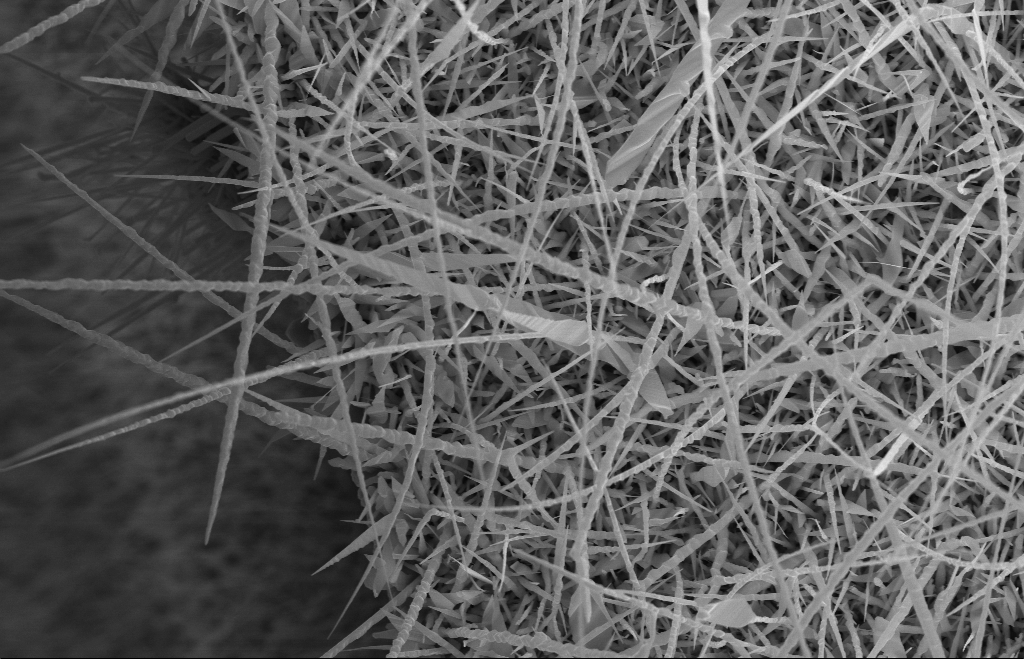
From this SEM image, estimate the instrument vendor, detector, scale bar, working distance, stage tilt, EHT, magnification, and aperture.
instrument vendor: Zeiss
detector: InLens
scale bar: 1000 nm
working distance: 9 mm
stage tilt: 0°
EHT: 10 kV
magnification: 20 K X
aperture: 30 µm